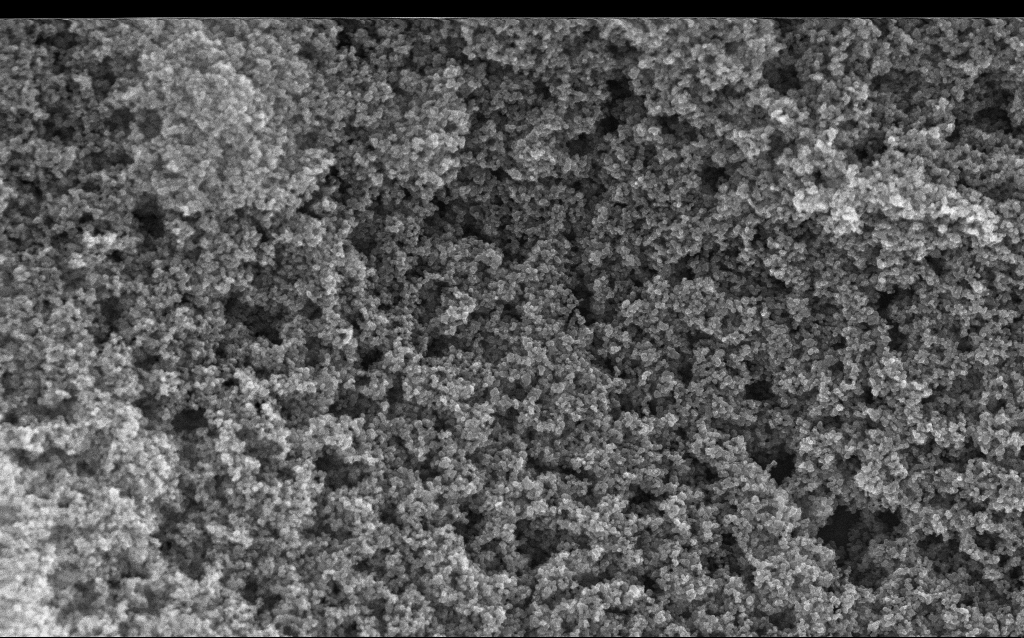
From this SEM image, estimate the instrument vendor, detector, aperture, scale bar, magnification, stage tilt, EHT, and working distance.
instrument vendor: Zeiss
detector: InLens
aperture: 30 µm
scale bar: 1000 nm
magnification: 65.04 K X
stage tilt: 0°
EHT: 10 kV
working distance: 2.4 mm